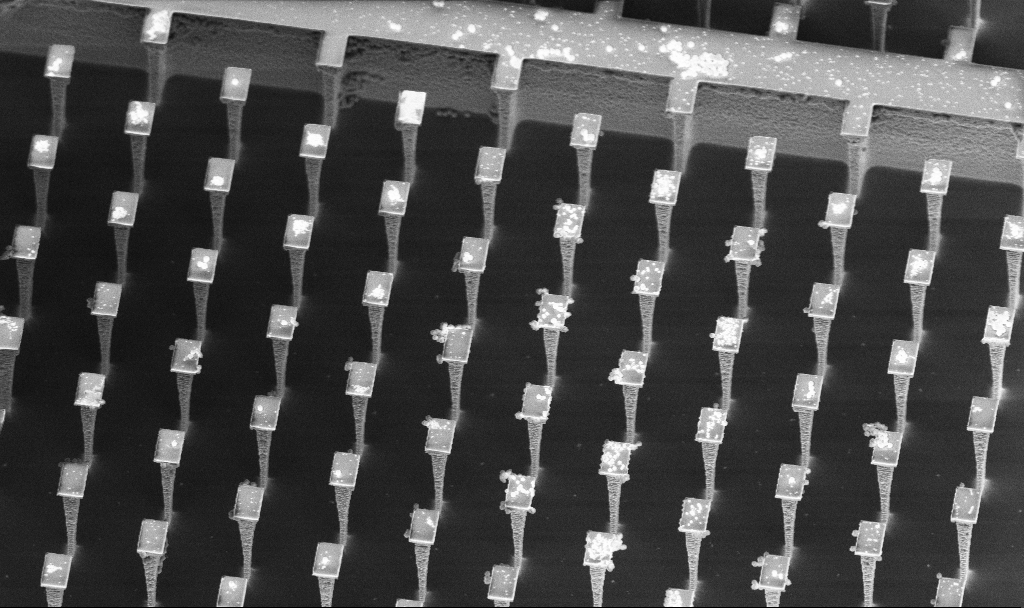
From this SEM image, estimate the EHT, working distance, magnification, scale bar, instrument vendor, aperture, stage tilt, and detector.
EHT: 5 kV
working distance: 5 mm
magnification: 3.25 K X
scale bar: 10000 nm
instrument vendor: Zeiss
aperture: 30 µm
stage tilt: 30°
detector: InLens